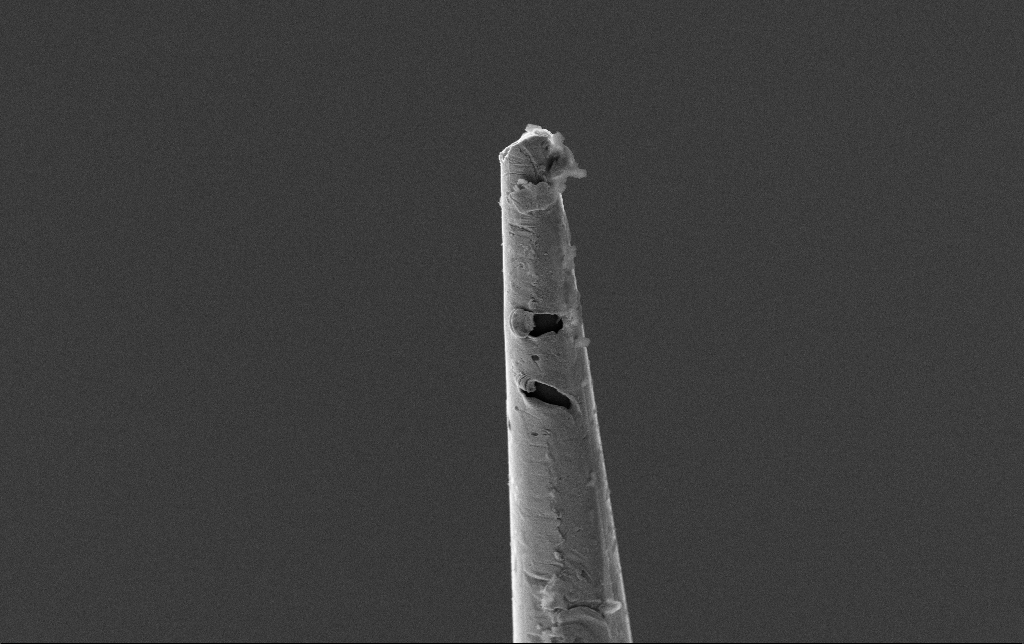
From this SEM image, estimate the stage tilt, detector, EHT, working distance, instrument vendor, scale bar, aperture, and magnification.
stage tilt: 27.9°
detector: SE2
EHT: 10 kV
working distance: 5.6 mm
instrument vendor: Zeiss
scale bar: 10000 nm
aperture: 30 µm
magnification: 4.41 K X